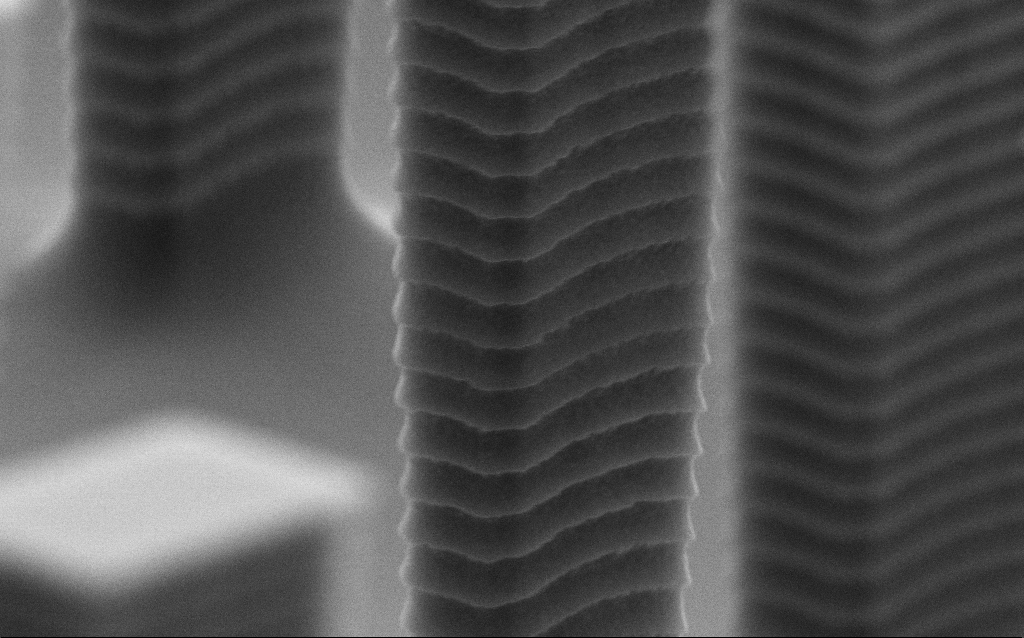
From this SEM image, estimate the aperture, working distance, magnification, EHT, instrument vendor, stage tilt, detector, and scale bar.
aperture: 30 µm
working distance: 6 mm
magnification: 50.67 K X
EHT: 6 kV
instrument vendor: Zeiss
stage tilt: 70°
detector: SE2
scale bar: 1000 nm